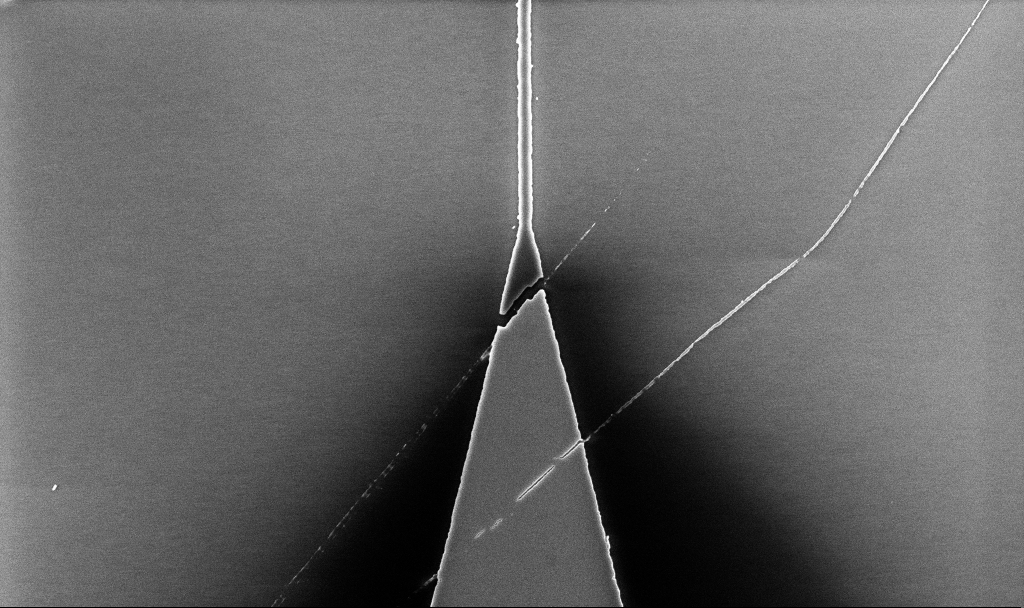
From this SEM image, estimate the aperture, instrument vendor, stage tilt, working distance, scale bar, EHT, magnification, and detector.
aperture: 30 µm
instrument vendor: Zeiss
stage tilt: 0°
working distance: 5.2 mm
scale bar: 2000 nm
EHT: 5 kV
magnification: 8.99 K X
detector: InLens